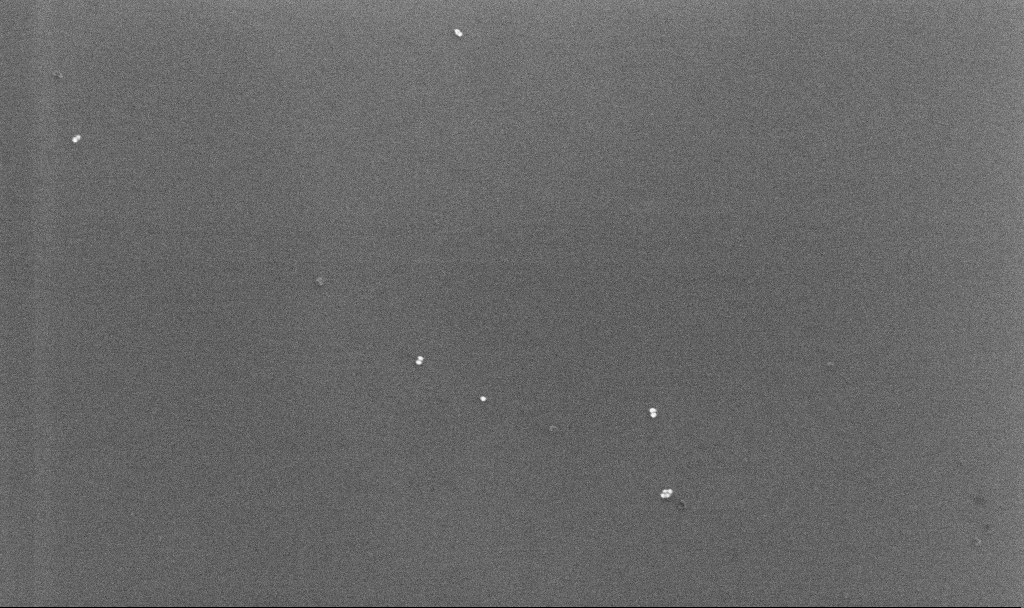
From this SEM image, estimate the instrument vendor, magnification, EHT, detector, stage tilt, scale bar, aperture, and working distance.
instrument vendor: Zeiss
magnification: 100 K X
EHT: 10 kV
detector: InLens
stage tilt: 0°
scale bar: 200 nm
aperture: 30 µm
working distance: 4.3 mm